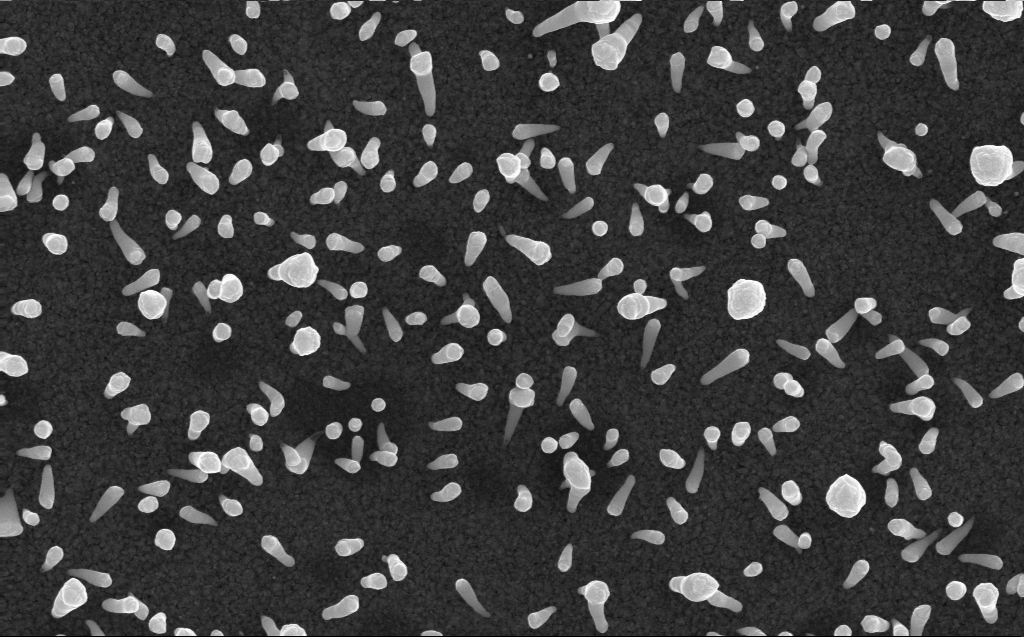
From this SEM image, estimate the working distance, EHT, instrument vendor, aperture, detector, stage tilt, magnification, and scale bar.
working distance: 3 mm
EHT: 10 kV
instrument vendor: Zeiss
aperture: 30 µm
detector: InLens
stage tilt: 0°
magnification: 50 K X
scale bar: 1000 nm